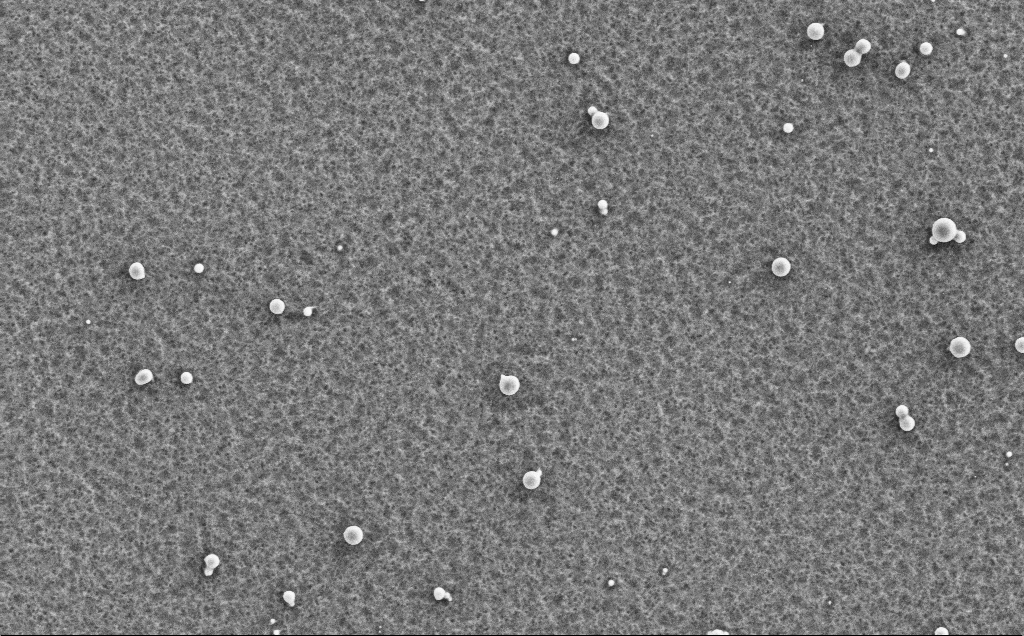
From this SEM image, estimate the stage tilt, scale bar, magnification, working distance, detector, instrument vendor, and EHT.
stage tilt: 0°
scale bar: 10000 nm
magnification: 5.58 K X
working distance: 5 mm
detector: SE2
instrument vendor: Zeiss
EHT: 5 kV